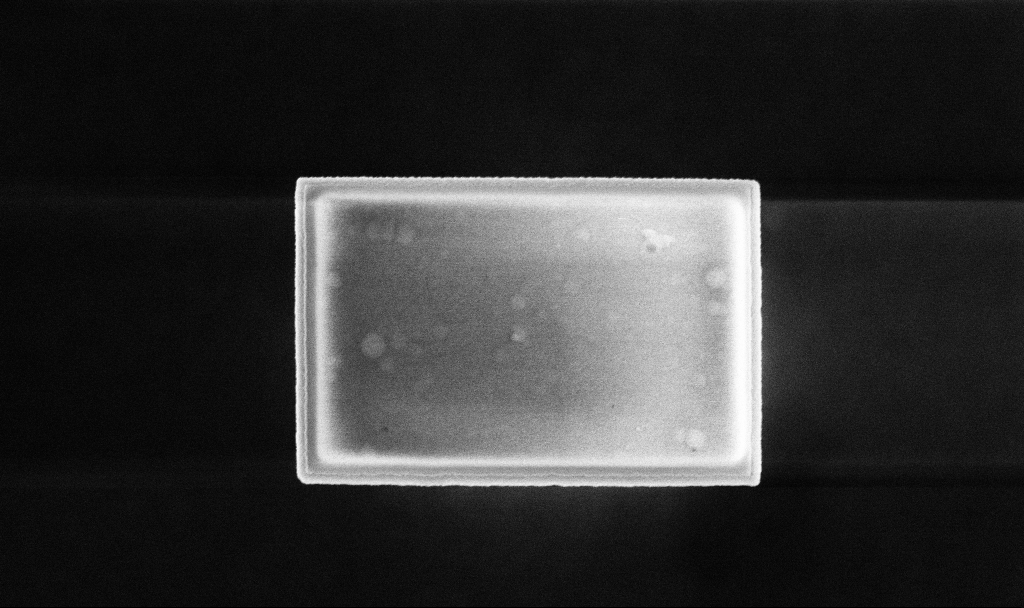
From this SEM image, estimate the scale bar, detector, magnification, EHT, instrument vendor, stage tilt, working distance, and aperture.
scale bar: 1000 nm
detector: InLens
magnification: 38.8 K X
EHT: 5 kV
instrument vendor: Zeiss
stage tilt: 0°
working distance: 3.3 mm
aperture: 30 µm